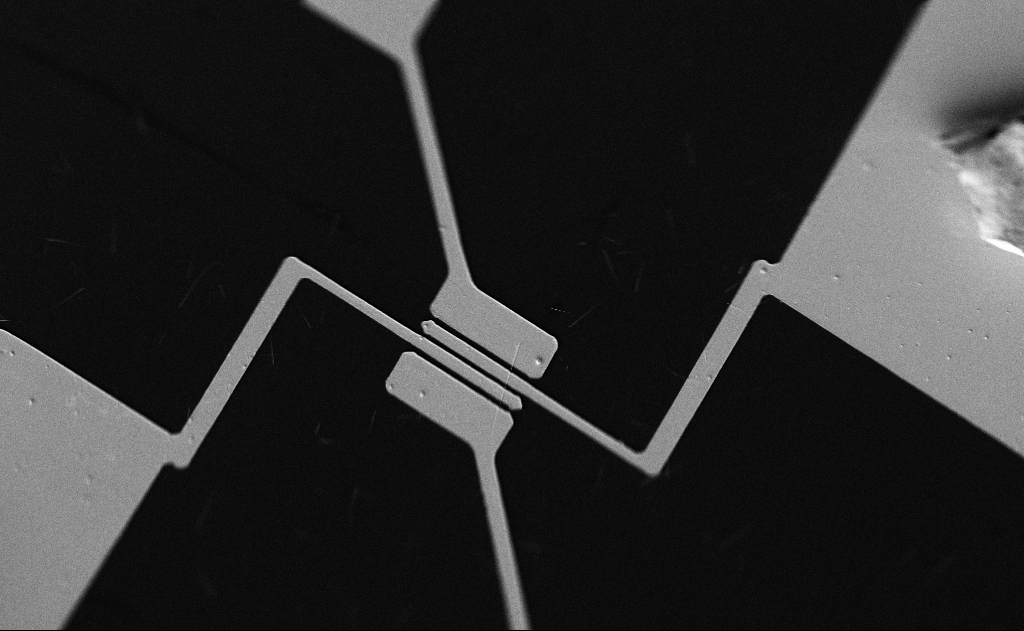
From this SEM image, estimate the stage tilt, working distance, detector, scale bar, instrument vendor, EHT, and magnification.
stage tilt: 0°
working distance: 21 mm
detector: SE2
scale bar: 10000 nm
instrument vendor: Zeiss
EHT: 5 kV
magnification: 1.58 K X